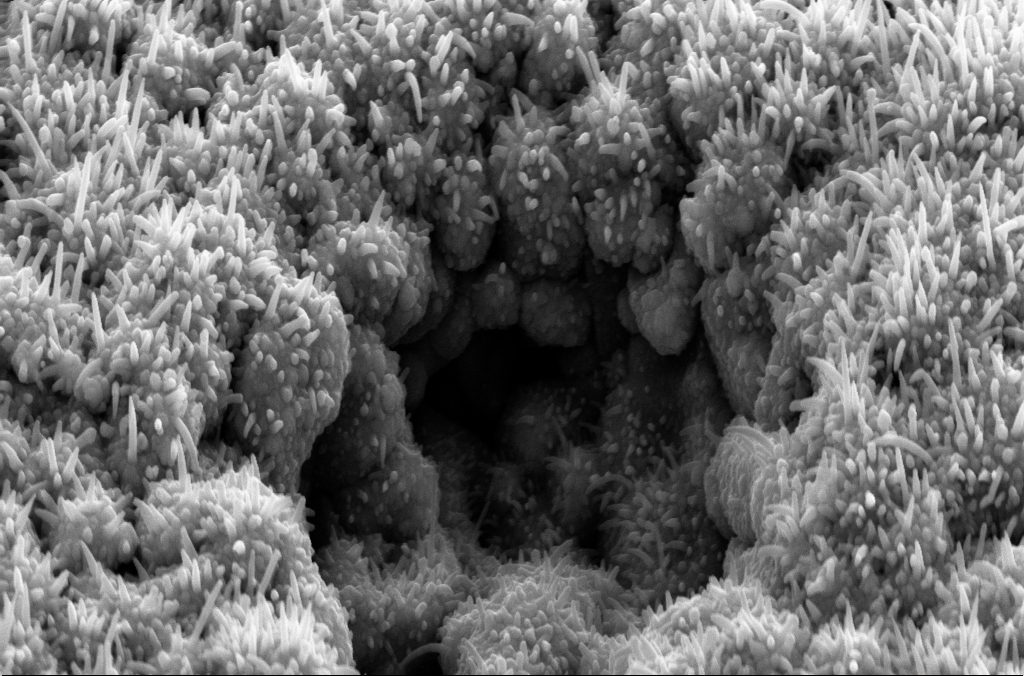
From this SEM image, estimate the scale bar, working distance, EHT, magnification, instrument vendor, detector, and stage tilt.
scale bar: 1000 nm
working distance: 8 mm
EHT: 10 kV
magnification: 44.05 K X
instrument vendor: Zeiss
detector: SE2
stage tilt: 45°